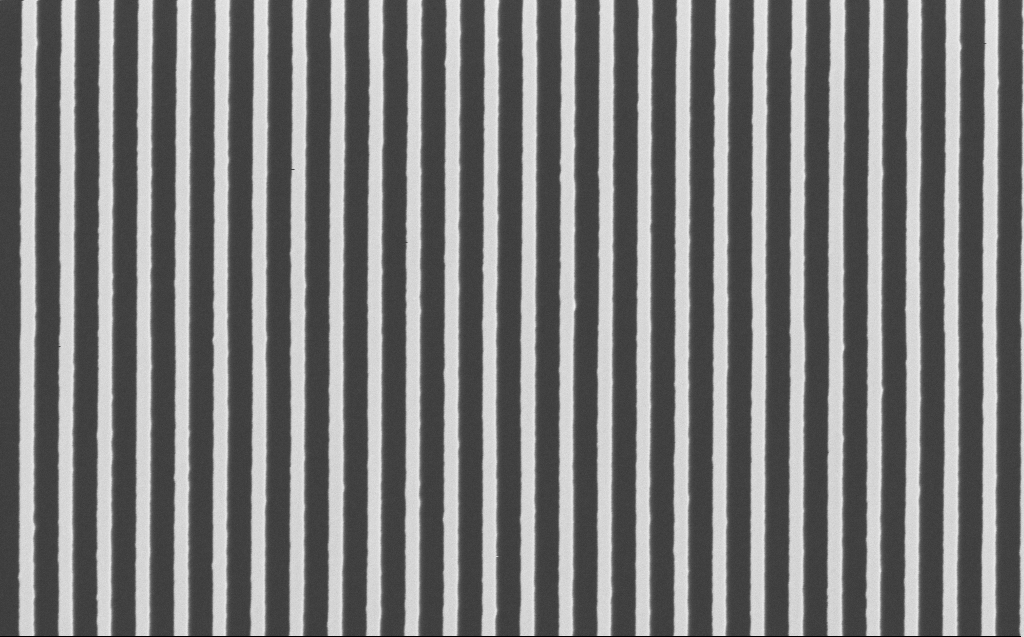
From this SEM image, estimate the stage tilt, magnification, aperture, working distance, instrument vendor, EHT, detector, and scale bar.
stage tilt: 0°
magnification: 40.33 K X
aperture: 30 µm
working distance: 7 mm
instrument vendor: Zeiss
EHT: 5 kV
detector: InLens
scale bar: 1000 nm